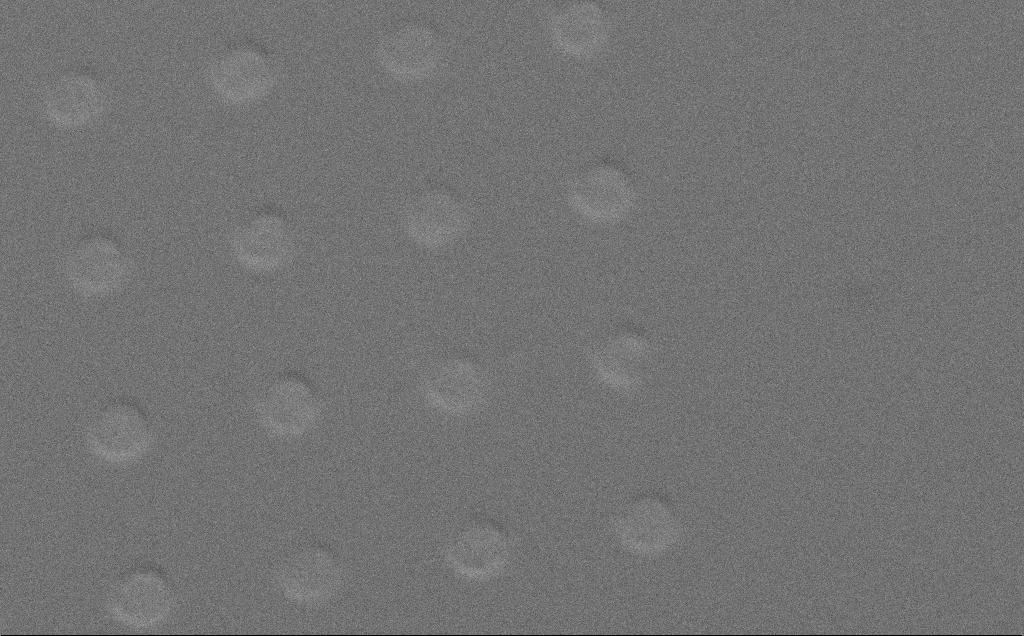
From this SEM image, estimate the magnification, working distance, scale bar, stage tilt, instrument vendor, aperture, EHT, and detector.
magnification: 2.86 K X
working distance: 6 mm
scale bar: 10000 nm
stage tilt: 40.4°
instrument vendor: Zeiss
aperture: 30 µm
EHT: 10 kV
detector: SE2